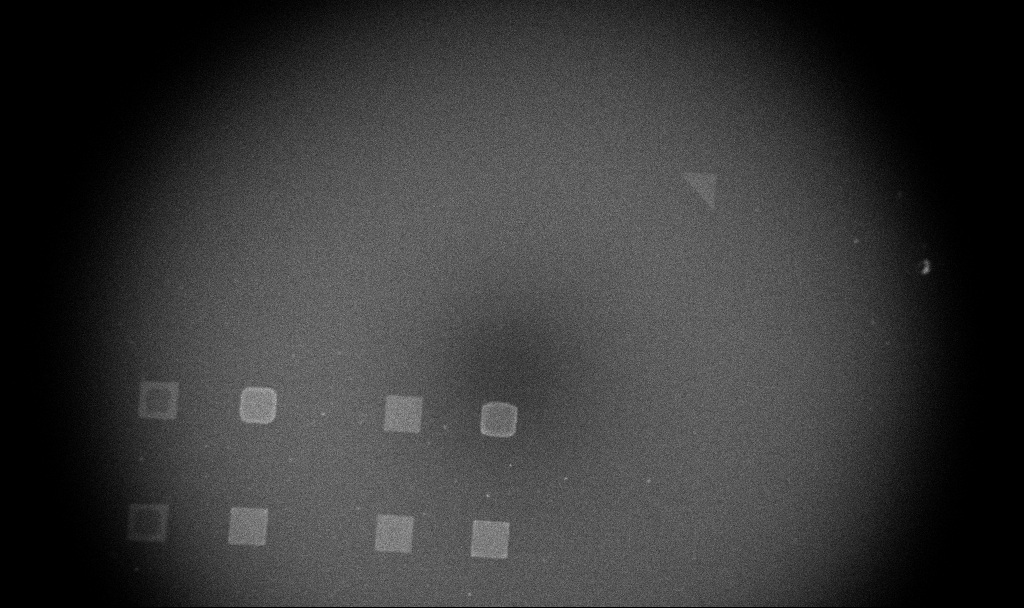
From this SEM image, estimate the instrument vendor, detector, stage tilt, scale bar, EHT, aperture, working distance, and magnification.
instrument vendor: Zeiss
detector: InLens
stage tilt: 0°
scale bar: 200000 nm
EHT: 5 kV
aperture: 30 µm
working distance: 4 mm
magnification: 0.09 K X